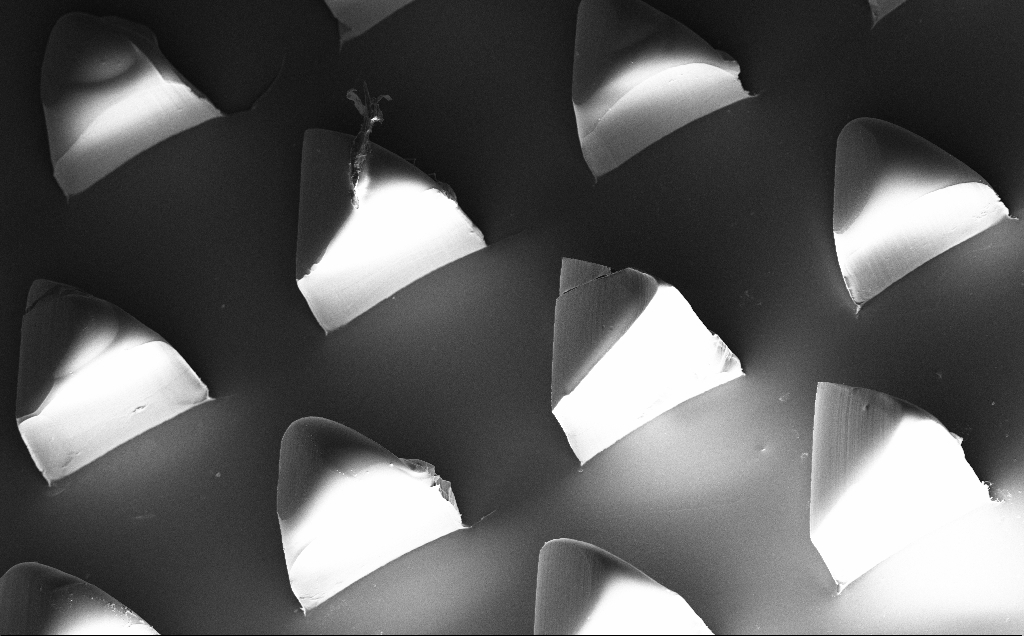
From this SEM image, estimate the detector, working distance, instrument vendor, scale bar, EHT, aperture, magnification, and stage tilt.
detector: InLens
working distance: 10 mm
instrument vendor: Zeiss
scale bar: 100000 nm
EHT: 10 kV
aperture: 30 µm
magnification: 0.254 K X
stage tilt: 20°